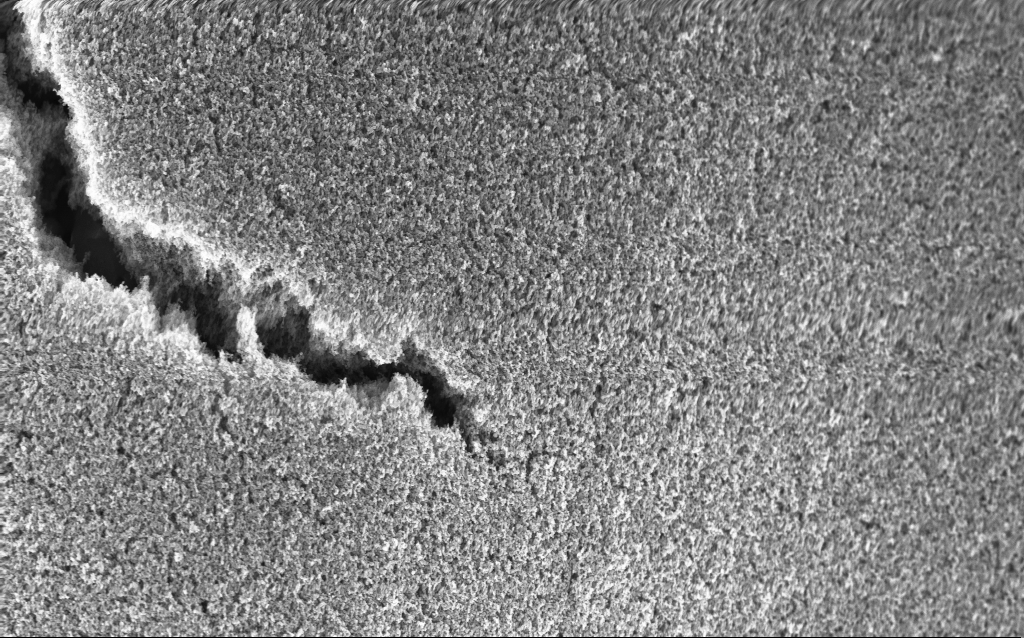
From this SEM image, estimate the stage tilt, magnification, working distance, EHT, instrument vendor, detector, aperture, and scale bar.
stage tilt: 0°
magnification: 37.8 K X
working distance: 2.6 mm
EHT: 5 kV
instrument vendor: Zeiss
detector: InLens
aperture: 30 µm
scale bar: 1000 nm